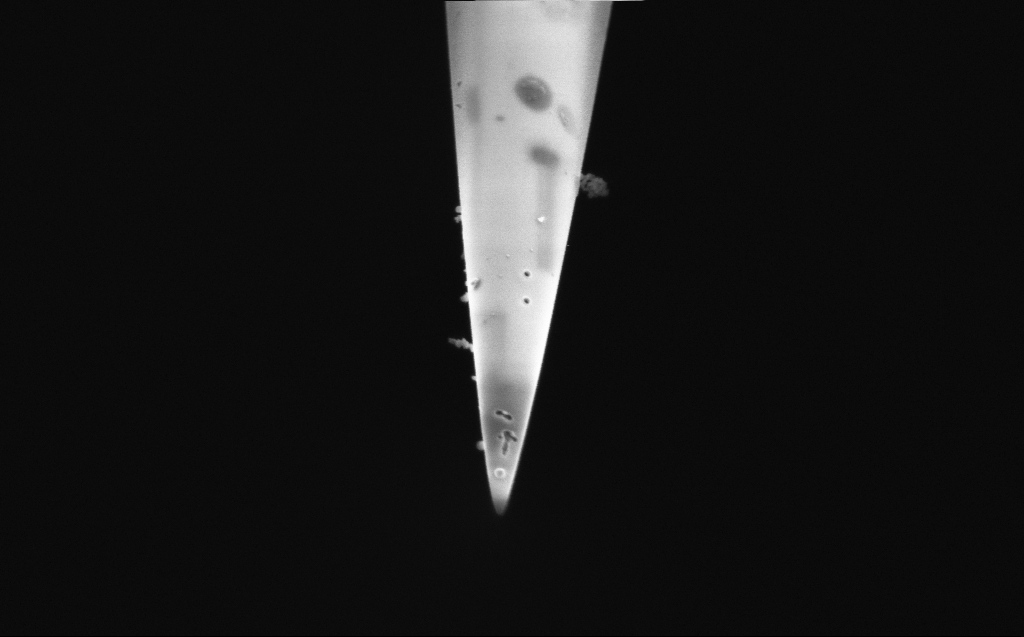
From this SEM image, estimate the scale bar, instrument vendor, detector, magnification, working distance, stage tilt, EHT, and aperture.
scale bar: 2000 nm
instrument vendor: Zeiss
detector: InLens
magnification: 35.29 K X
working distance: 3 mm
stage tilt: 45.1°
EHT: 2 kV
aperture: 20 µm